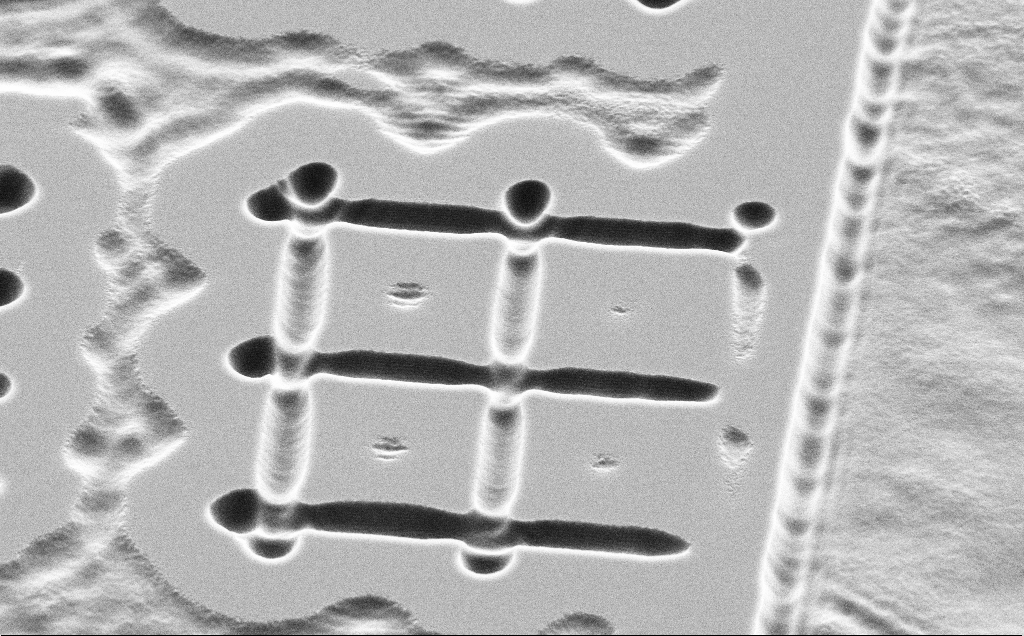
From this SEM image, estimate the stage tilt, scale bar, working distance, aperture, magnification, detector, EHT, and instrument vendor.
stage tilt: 45°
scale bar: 2000 nm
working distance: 11 mm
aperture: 30 µm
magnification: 7.86 K X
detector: SE2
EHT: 5 kV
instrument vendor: Zeiss